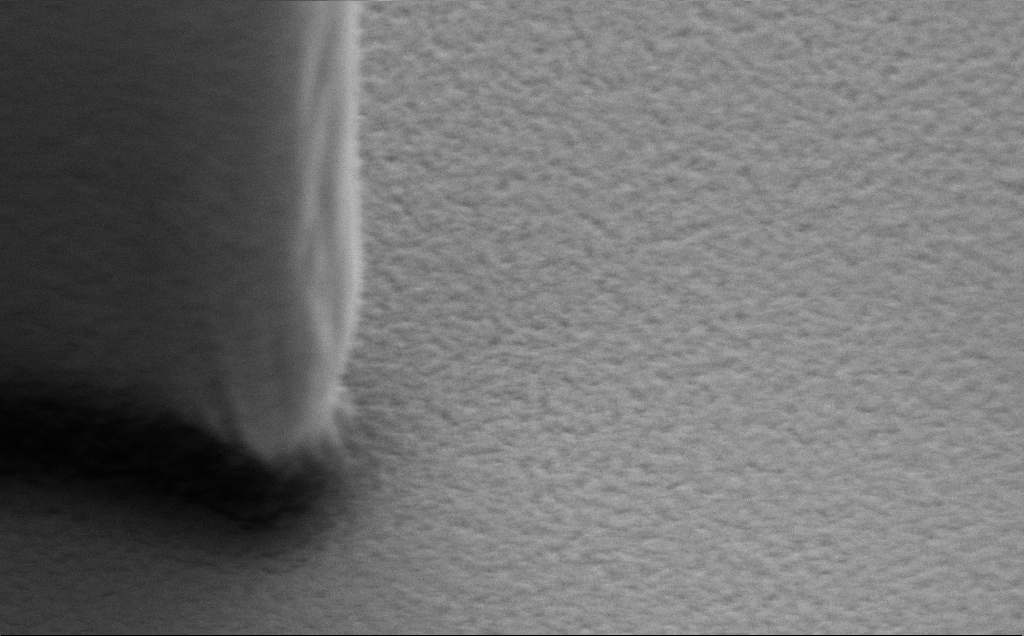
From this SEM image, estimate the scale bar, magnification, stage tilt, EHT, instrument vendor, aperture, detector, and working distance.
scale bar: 1000 nm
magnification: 57.76 K X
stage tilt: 40°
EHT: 5 kV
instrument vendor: Zeiss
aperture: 30 µm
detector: SE2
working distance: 9 mm